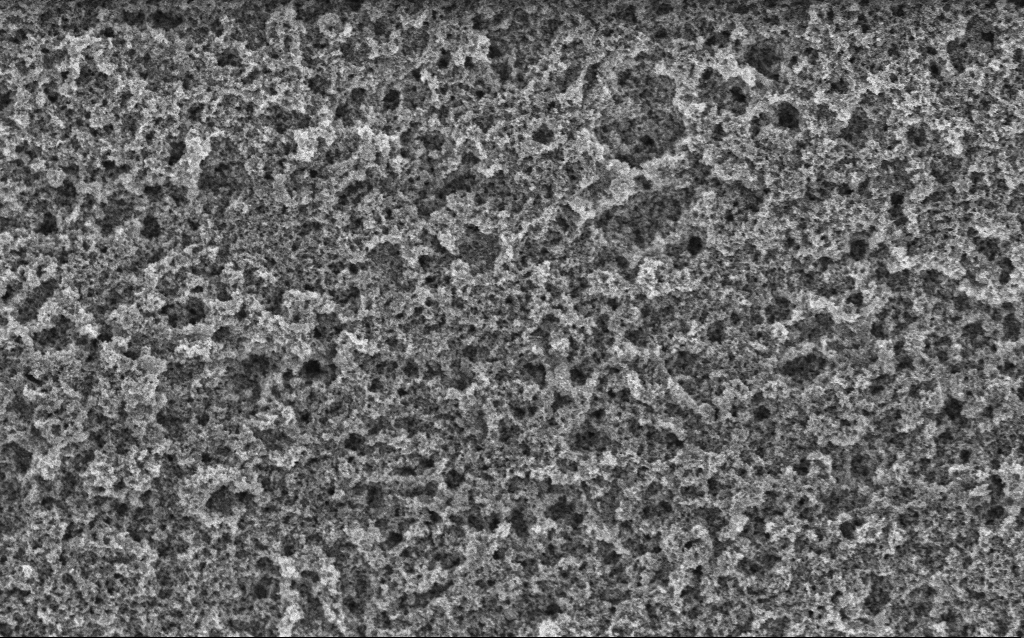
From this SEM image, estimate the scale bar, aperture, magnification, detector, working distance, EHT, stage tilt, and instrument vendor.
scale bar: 1000 nm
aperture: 30 µm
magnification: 15.33 K X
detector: InLens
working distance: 4.4 mm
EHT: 5 kV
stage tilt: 0°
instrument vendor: Zeiss